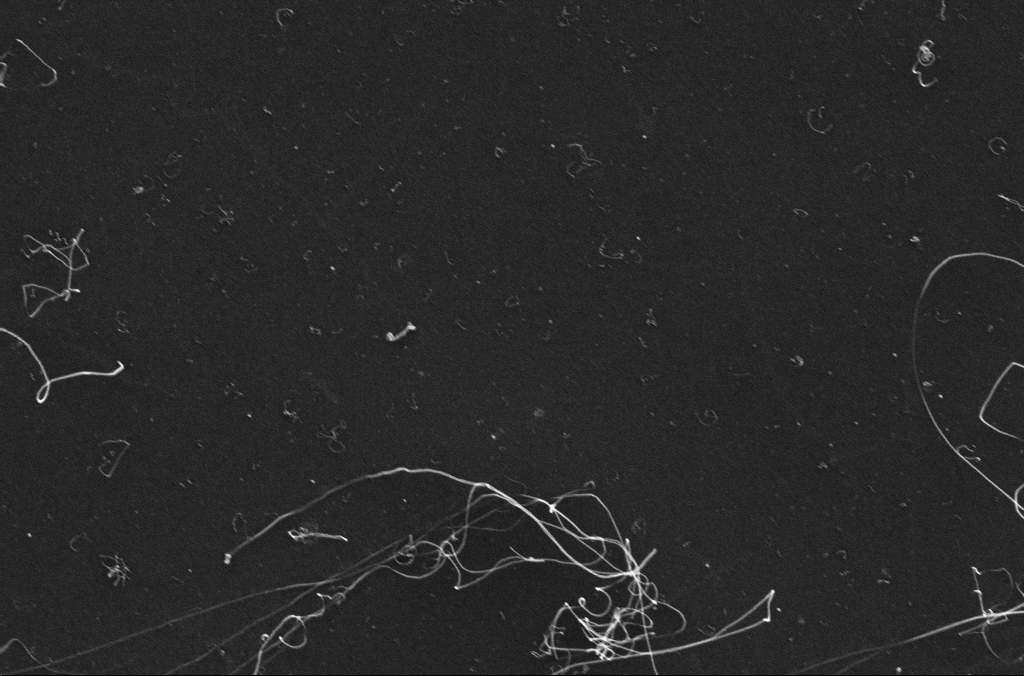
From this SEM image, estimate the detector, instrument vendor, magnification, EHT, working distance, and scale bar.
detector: InLens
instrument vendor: Zeiss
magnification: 50 K X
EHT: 10 kV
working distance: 3.3 mm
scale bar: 1000 nm